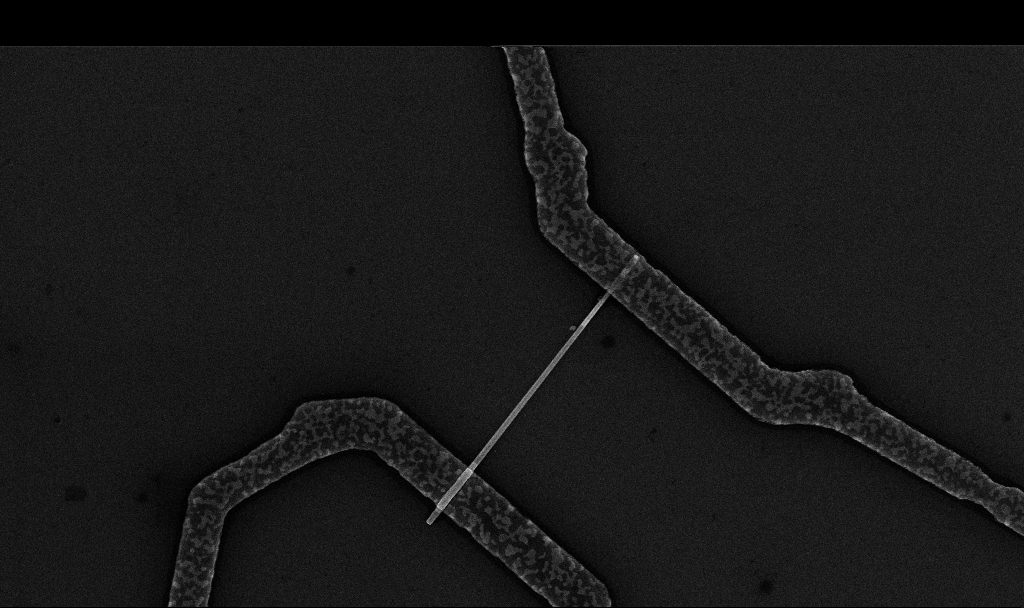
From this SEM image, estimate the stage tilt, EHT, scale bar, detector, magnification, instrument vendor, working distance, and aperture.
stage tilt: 0°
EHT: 10 kV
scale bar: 1000 nm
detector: InLens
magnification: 20.34 K X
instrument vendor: Zeiss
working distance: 6.7 mm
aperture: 30 µm